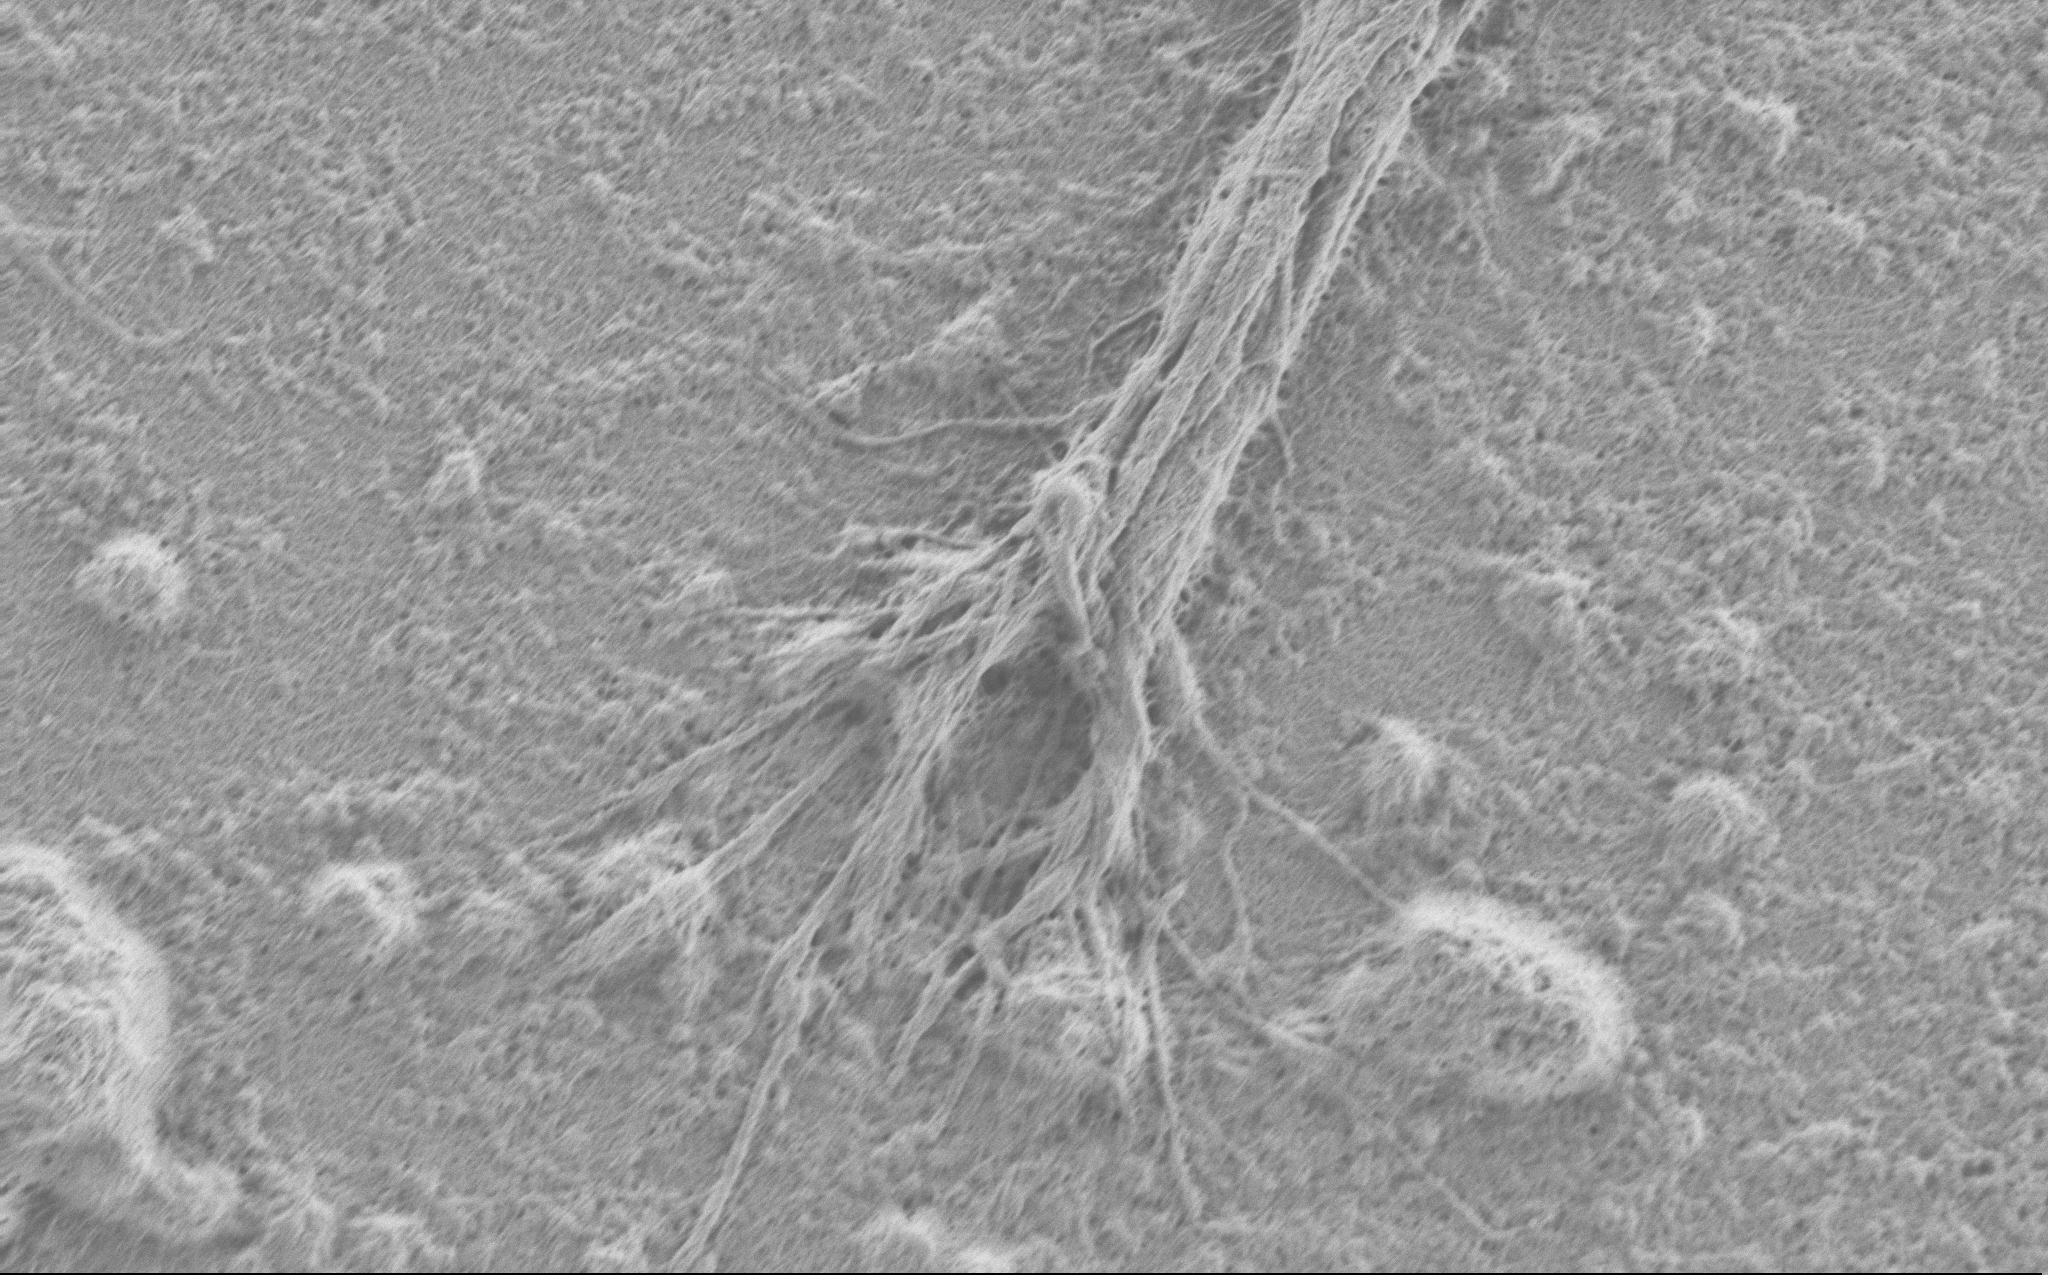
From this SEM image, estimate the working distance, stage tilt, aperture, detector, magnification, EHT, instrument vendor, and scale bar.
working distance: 4 mm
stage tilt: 0°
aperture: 30 µm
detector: SE2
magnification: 10 K X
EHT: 0.9 kV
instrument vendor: Zeiss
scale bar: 2000 nm